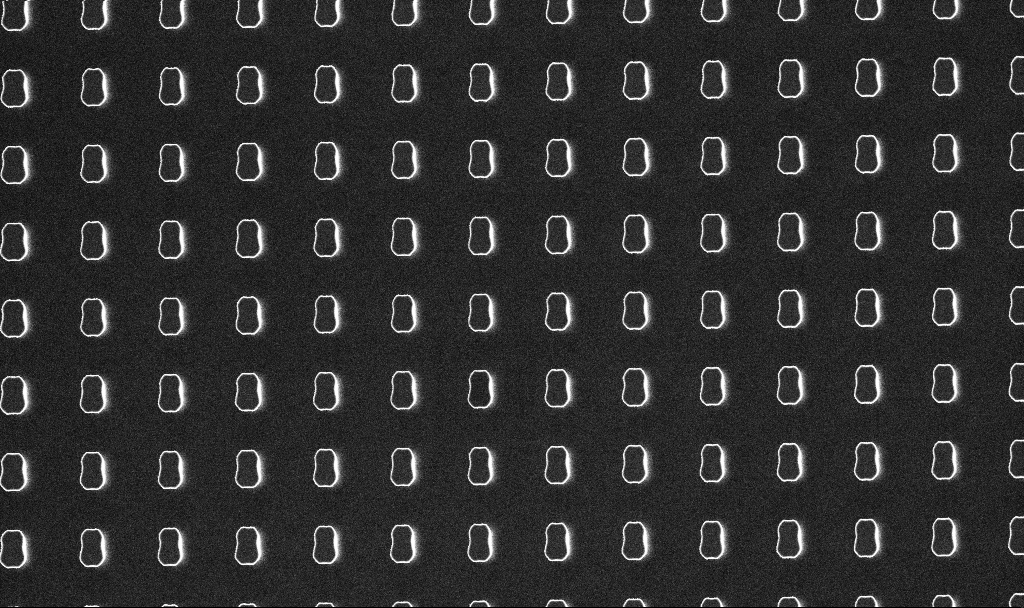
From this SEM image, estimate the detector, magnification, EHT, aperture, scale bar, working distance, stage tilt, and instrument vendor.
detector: InLens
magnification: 4.72 K X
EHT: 3 kV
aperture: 30 µm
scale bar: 10000 nm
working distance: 7.5 mm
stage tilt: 0°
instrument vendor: Zeiss